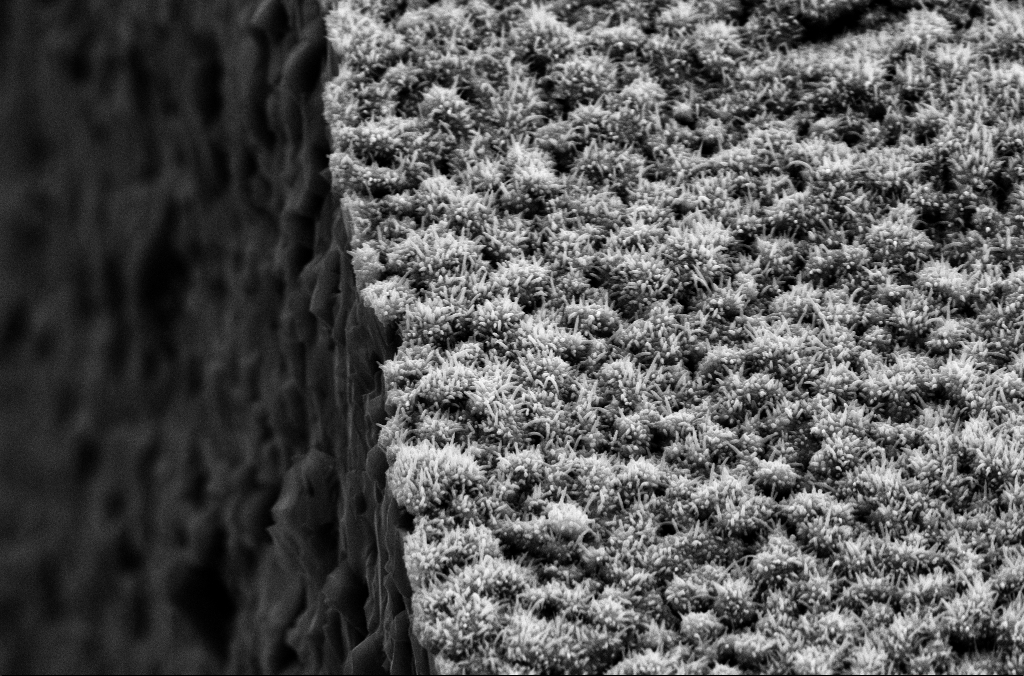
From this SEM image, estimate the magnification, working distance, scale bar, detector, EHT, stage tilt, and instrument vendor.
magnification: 20.48 K X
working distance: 6.6 mm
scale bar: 1000 nm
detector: SE2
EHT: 10 kV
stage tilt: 45°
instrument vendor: Zeiss